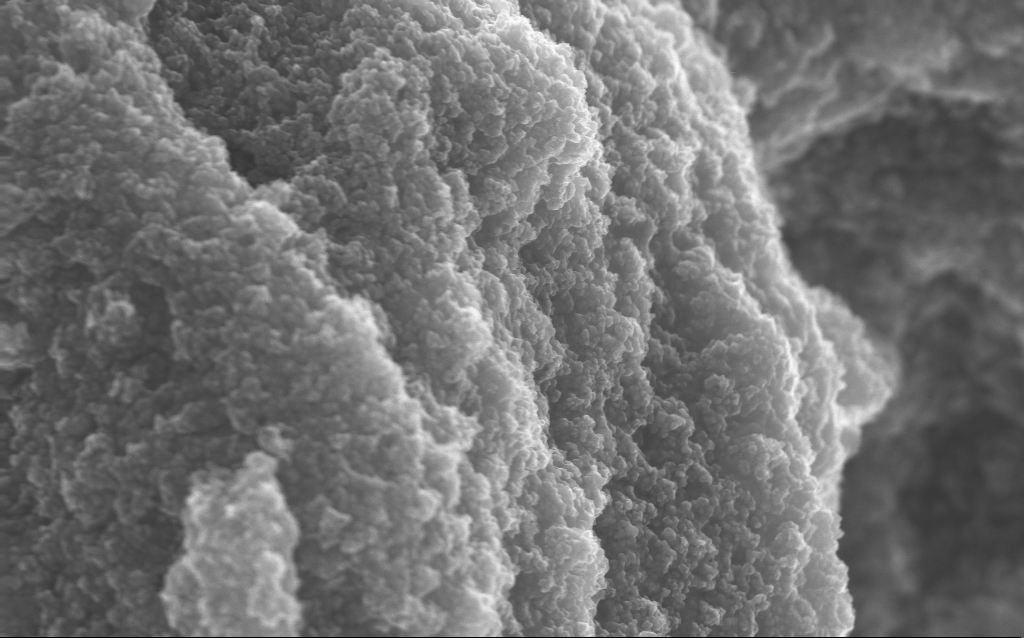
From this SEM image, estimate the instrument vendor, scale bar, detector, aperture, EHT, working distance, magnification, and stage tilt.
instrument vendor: Zeiss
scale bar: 1000 nm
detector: InLens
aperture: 30 µm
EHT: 10 kV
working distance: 2.7 mm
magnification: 65.04 K X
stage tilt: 0°